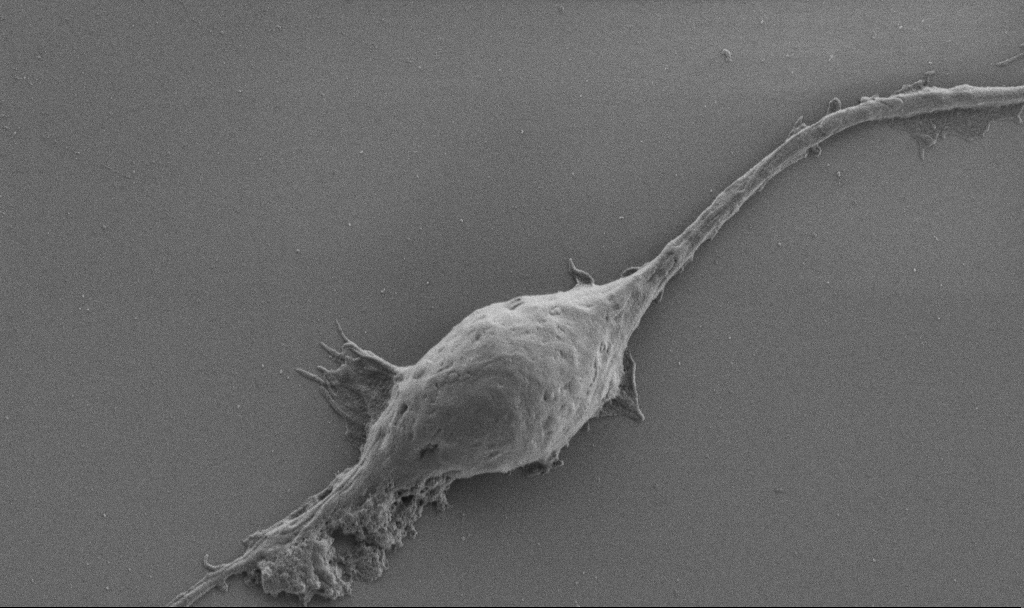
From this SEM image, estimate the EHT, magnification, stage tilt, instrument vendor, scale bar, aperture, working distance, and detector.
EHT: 1 kV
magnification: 10 K X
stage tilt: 0°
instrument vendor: Zeiss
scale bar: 2000 nm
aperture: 30 µm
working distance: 6.9 mm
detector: SE2